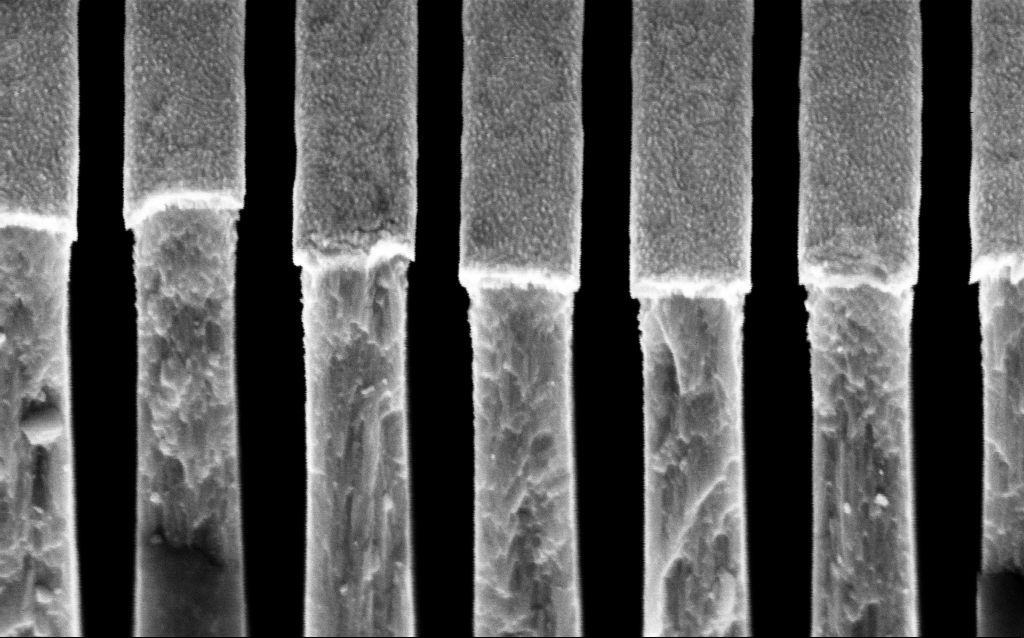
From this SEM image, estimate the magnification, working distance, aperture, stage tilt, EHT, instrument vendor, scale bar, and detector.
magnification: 125.27 K X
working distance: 6 mm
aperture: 30 µm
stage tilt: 45°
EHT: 3 kV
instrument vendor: Zeiss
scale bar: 200 nm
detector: InLens